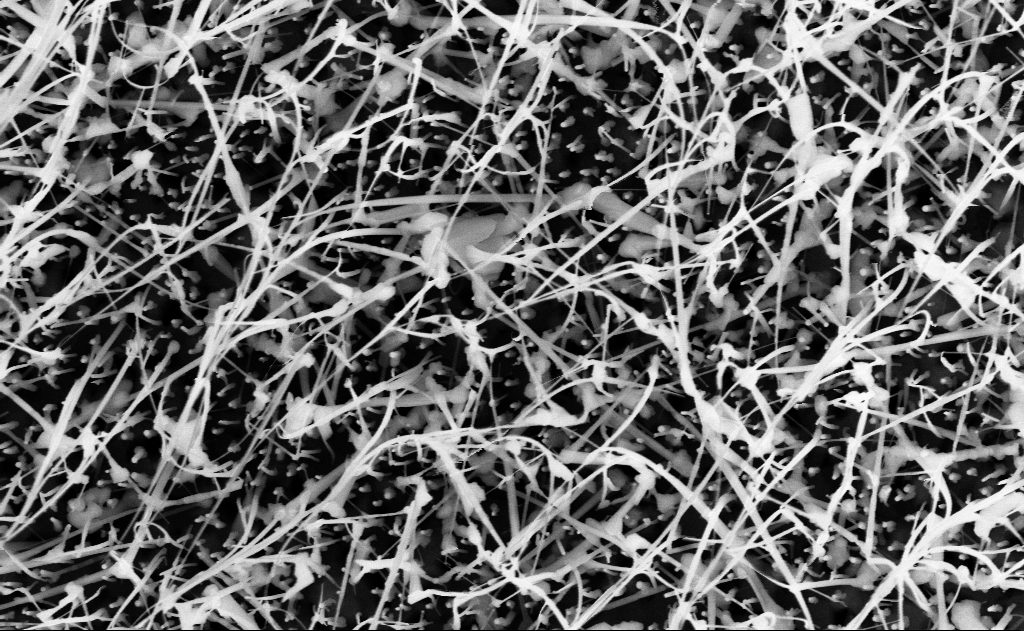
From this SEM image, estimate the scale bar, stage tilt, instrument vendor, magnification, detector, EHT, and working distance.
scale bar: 1000 nm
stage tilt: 0°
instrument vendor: Zeiss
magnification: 40 K X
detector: InLens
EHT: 10 kV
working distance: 14 mm